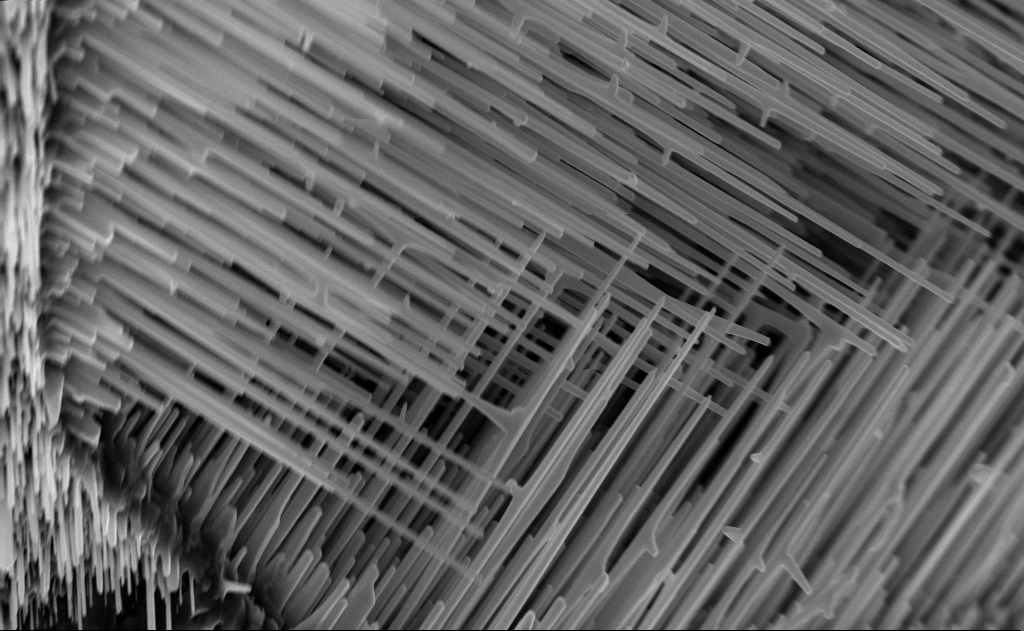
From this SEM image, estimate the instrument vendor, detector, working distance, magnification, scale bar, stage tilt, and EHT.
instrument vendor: Zeiss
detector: InLens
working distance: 6 mm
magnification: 20 K X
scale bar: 2000 nm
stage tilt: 0°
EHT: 10 kV